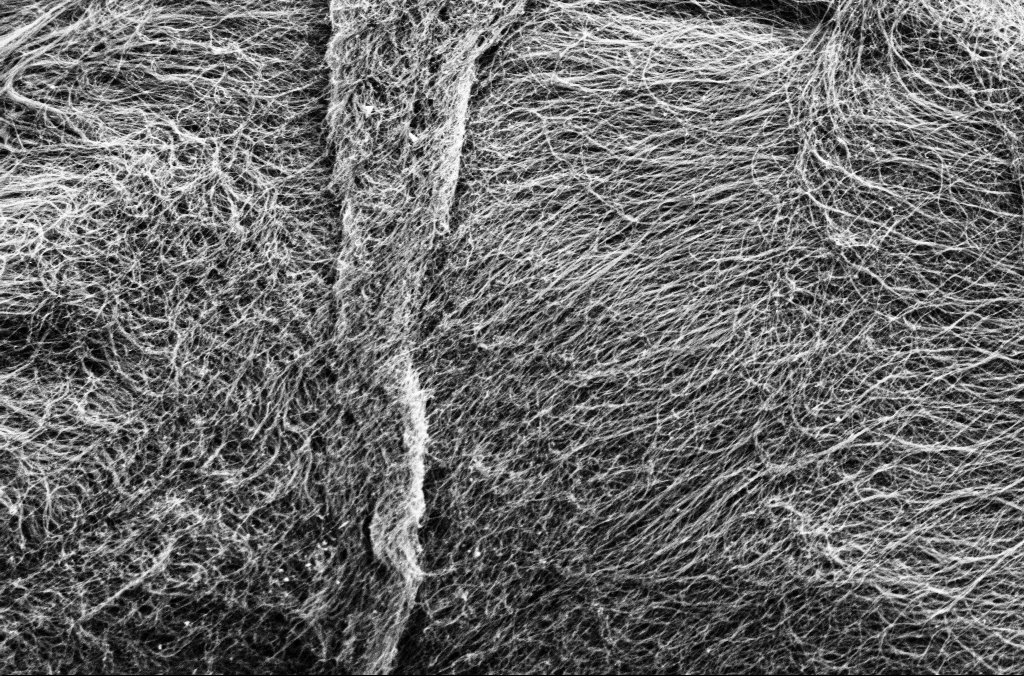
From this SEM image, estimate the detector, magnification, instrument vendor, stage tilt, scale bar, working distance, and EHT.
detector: SE2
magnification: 25 K X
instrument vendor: Zeiss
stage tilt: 0°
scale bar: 1000 nm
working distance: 5.4 mm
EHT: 1.8 kV